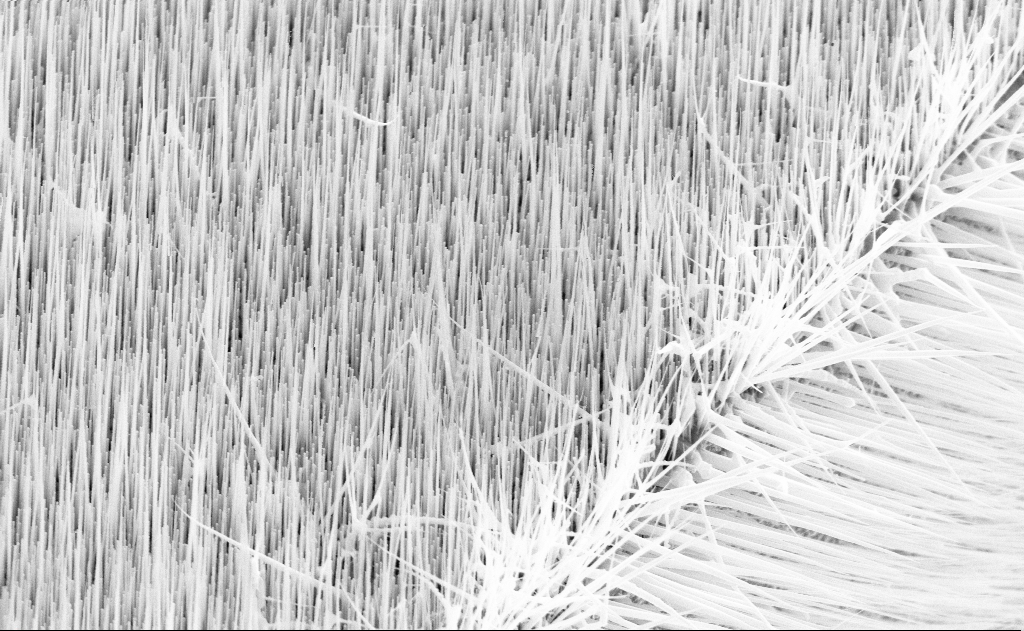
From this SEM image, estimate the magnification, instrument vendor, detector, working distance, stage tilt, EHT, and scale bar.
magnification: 20 K X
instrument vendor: Zeiss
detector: SE2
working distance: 16 mm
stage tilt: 45°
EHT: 10 kV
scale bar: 2000 nm